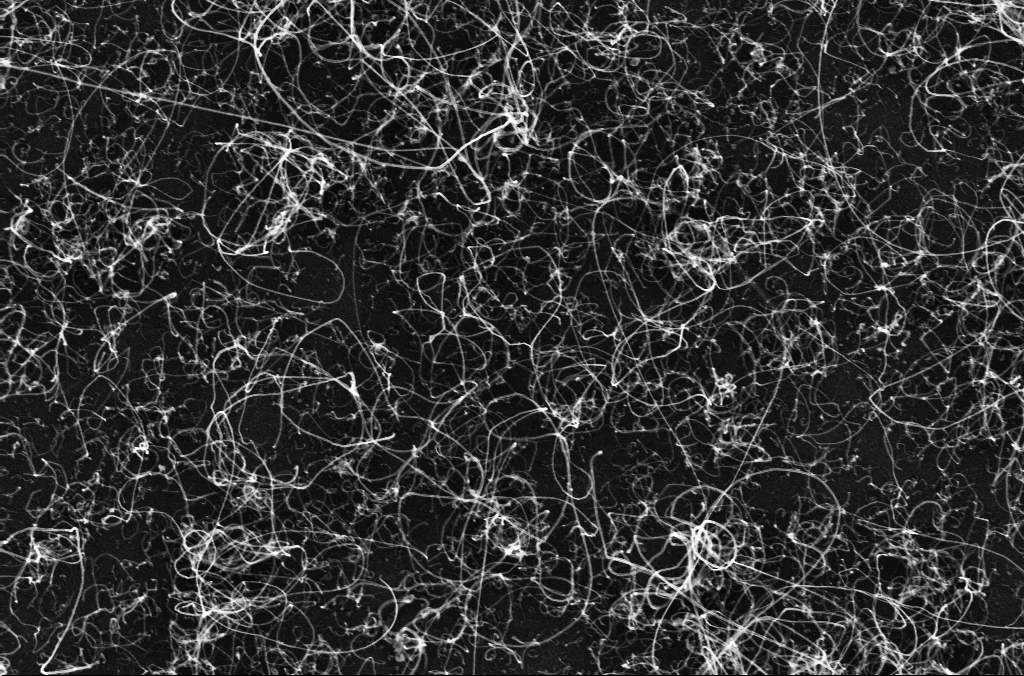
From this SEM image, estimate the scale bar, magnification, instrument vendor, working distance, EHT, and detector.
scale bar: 1000 nm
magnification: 50 K X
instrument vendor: Zeiss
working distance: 3.3 mm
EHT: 10 kV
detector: InLens